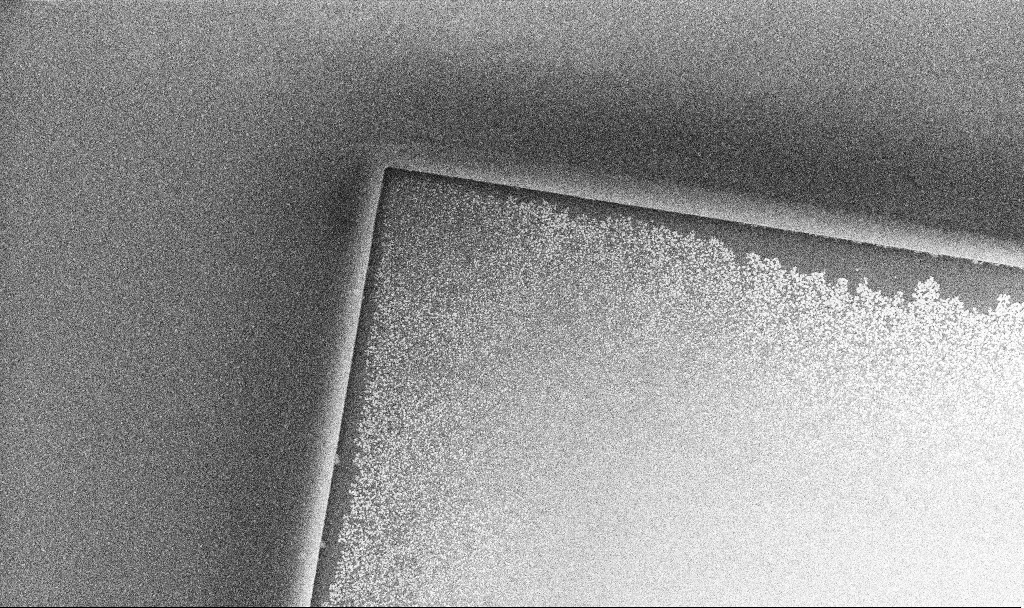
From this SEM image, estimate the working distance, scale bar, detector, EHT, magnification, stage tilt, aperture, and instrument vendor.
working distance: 5.8 mm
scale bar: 1000 nm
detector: InLens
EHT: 5 kV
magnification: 24.59 K X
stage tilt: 0°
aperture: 30 µm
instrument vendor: Zeiss